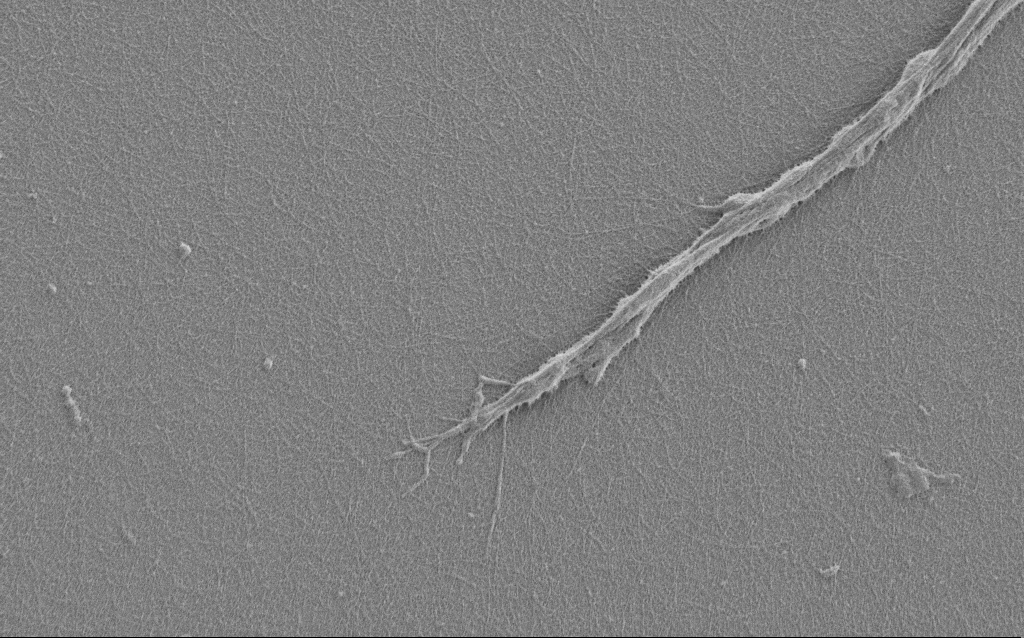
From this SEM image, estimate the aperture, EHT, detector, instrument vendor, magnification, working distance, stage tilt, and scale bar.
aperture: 30 µm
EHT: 1 kV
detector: SE2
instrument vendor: Zeiss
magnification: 7.5 K X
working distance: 6 mm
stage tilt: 0°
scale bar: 2000 nm